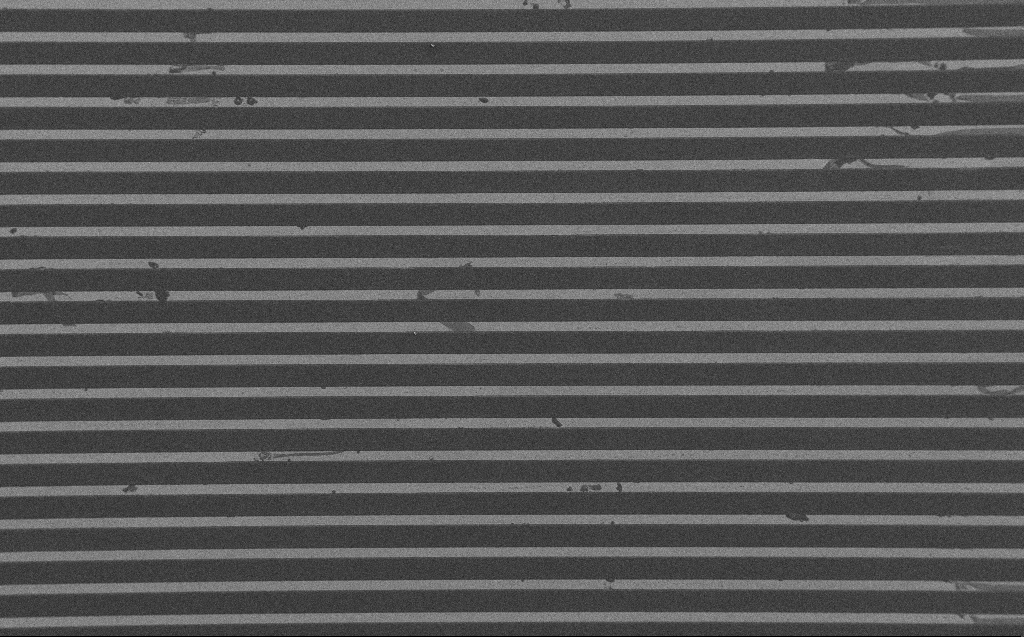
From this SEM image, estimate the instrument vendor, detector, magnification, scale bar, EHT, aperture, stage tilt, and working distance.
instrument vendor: Zeiss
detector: SE2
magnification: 0.434 K X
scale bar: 100000 nm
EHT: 1.2 kV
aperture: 30 µm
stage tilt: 0°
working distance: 4 mm